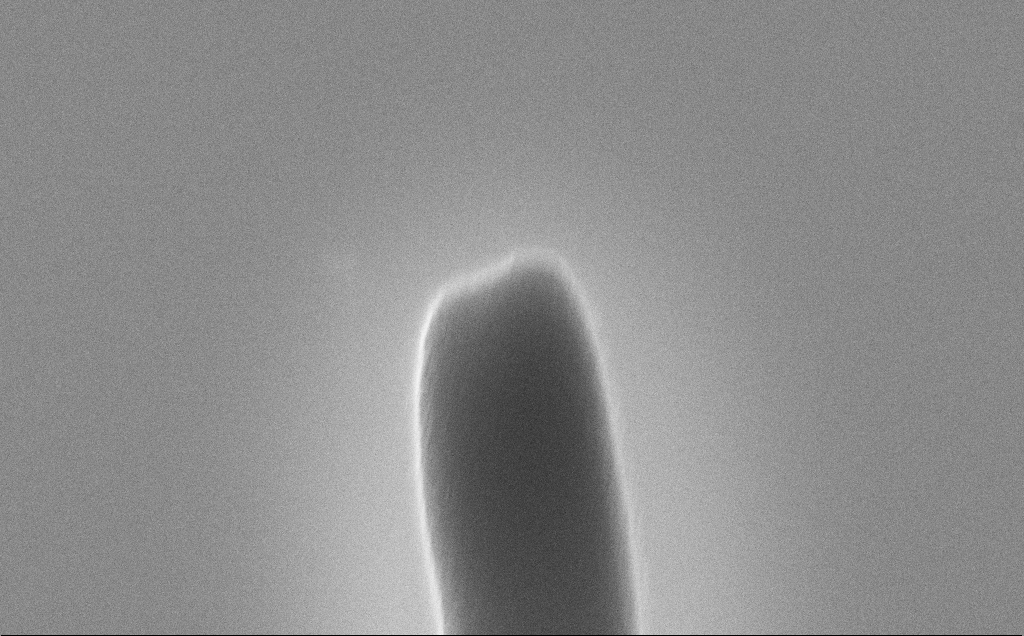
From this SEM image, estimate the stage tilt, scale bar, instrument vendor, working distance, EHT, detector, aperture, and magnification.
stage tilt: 0°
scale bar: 1000 nm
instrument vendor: Zeiss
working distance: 15 mm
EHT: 10 kV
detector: SE2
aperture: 30 µm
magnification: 53.71 K X